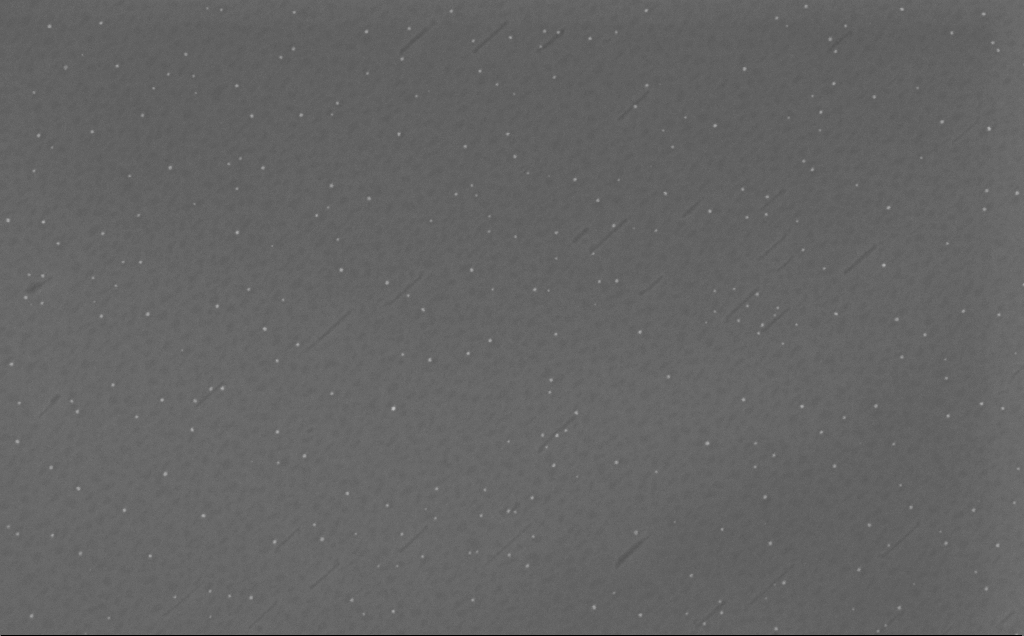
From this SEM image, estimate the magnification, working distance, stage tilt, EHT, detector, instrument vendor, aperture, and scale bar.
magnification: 154.79 K X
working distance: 6 mm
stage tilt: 0°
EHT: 10 kV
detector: InLens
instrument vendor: Zeiss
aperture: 30 µm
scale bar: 100 nm